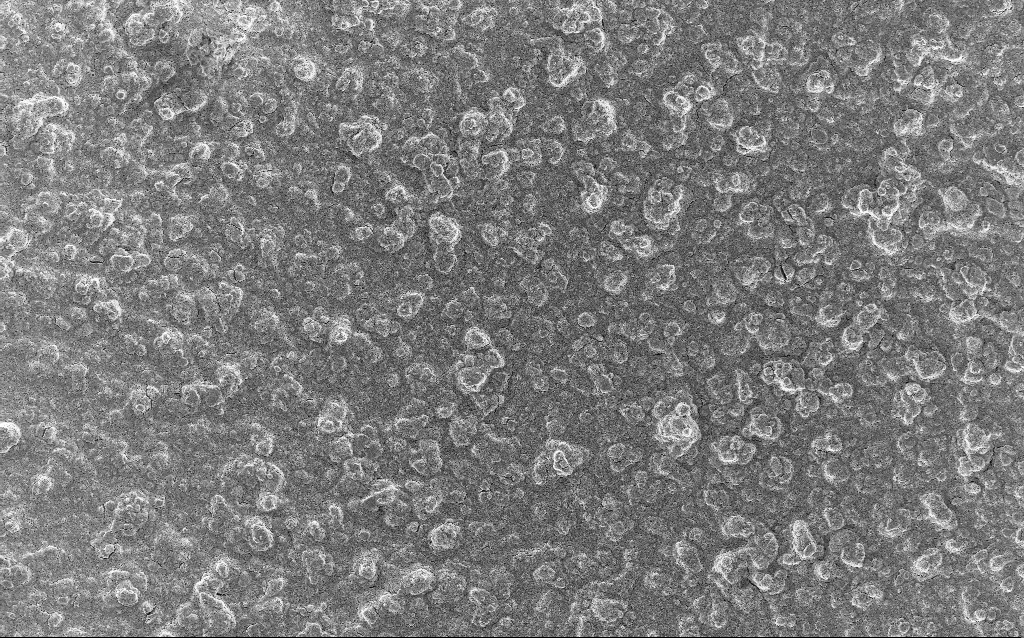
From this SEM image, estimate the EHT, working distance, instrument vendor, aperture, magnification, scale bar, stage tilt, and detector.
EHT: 5 kV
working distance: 3 mm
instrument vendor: Zeiss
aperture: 30 µm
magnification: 0.77 K X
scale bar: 20000 nm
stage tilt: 0°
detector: InLens